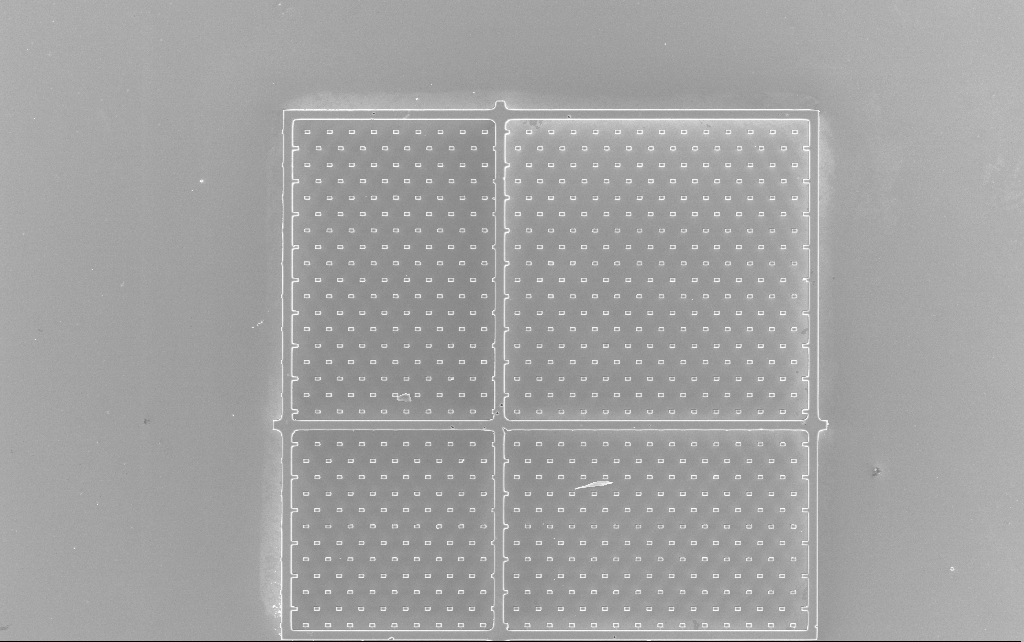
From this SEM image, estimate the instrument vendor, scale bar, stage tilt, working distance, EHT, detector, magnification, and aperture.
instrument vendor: Zeiss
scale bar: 100000 nm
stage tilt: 0°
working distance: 2.5 mm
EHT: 10 kV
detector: InLens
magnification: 0.676 K X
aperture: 30 µm